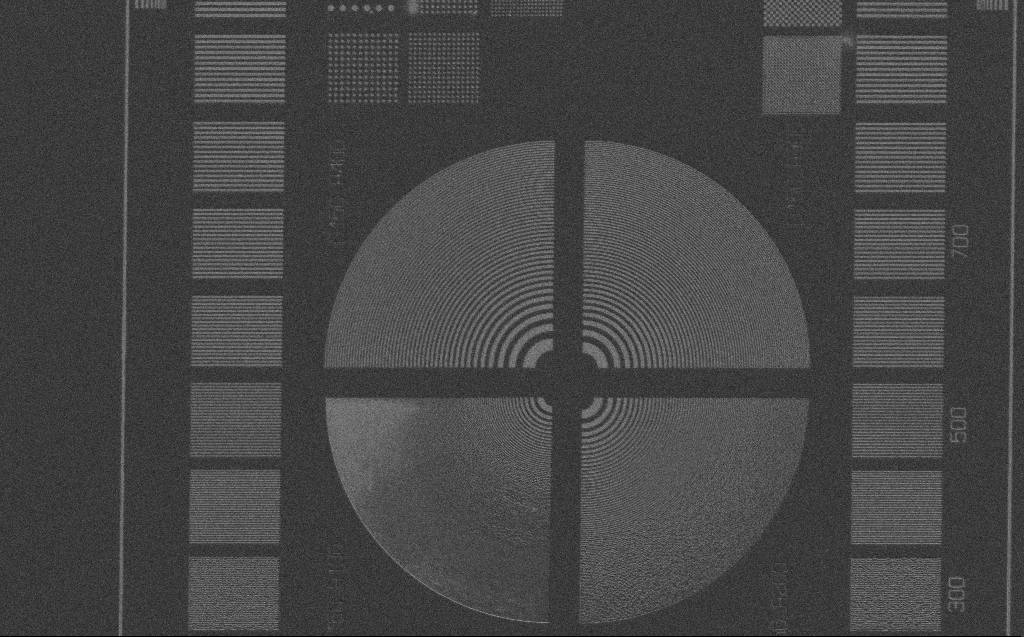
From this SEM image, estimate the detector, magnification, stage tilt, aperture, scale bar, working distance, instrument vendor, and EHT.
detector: SE2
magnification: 1.12 K X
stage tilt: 0°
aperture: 30 µm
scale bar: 20000 nm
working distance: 4 mm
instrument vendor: Zeiss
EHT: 3 kV